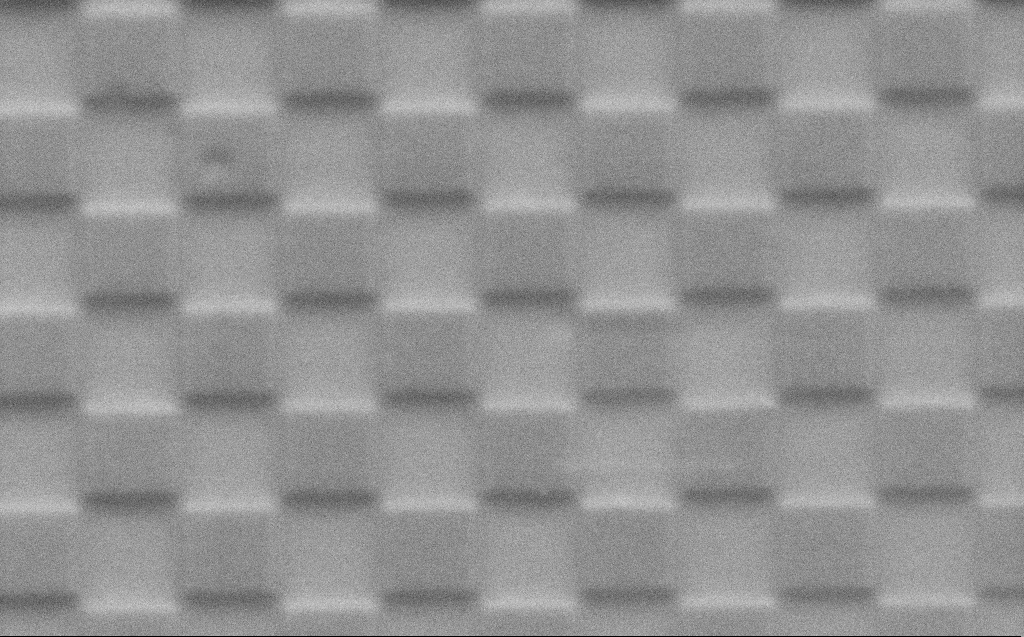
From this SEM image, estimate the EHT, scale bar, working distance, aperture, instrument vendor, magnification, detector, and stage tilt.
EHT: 2 kV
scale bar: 1000 nm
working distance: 4 mm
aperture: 30 µm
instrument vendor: Zeiss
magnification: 37.3 K X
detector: SE2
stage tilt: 45°